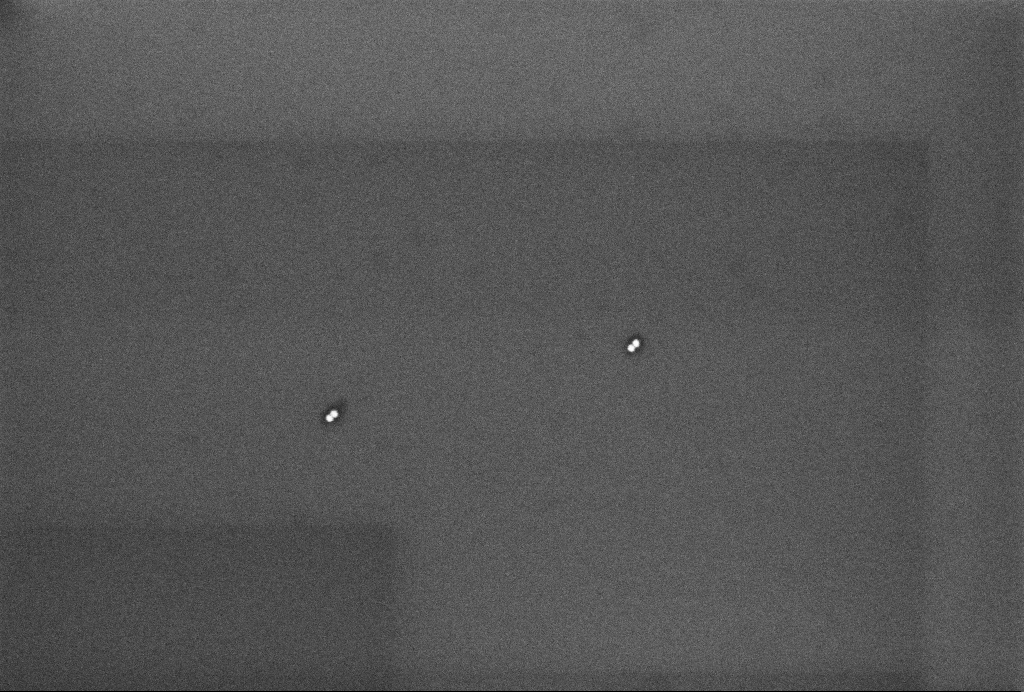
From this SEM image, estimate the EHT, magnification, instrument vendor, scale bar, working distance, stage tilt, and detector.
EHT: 3 kV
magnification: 104.07 K X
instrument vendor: Zeiss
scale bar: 200 nm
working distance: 3.2 mm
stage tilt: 0°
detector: InLens